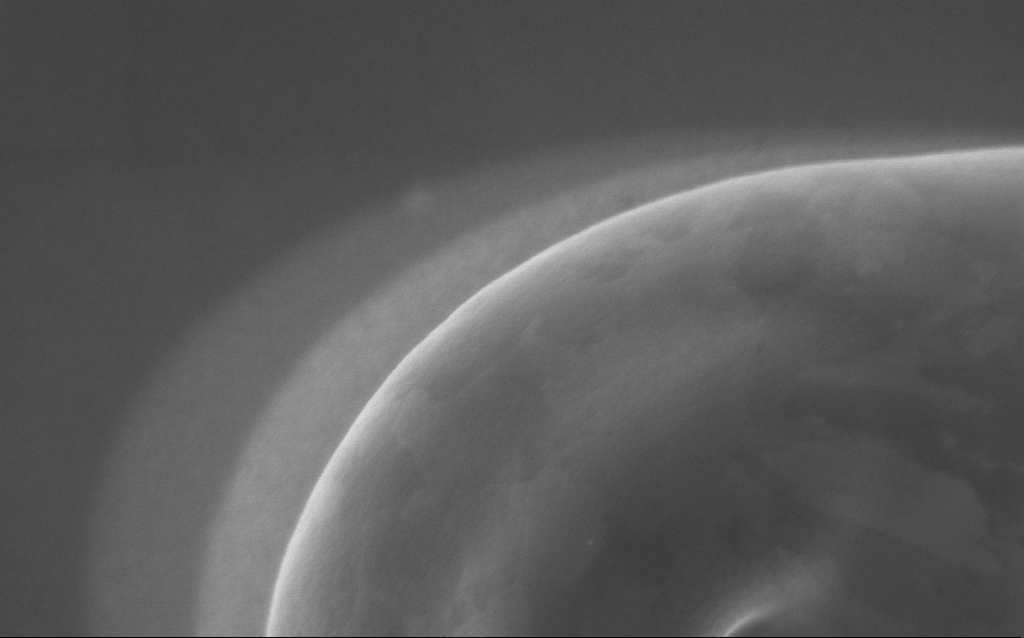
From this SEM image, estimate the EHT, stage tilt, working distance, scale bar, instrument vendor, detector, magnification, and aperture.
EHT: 5 kV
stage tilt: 0°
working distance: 3 mm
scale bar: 200 nm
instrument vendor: Zeiss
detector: InLens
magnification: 99.94 K X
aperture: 30 µm